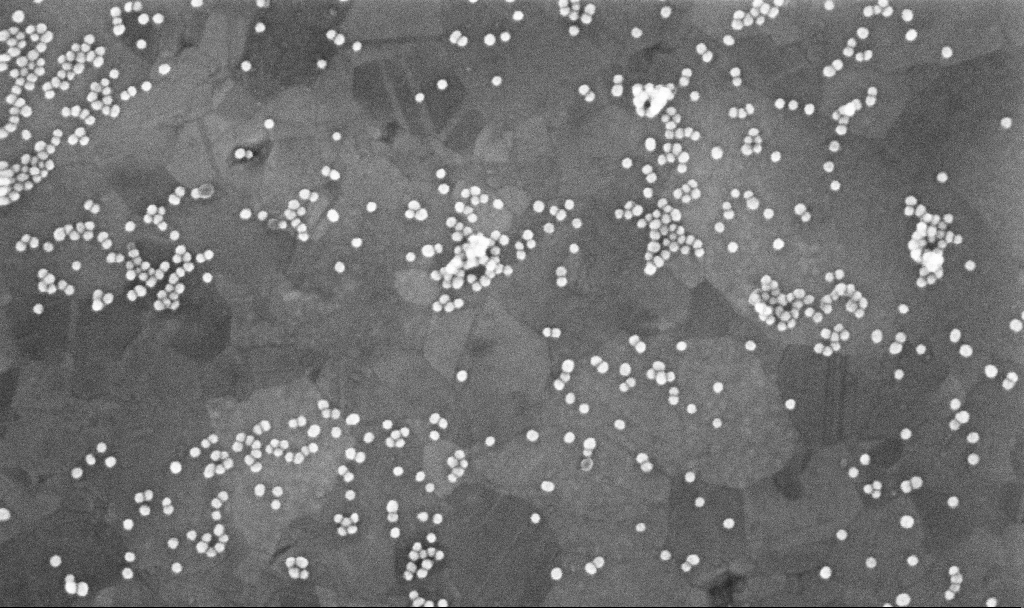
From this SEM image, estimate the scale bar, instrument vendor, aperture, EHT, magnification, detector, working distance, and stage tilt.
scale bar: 100 nm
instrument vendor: Zeiss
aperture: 30 µm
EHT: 10 kV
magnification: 200 K X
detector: InLens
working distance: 3.7 mm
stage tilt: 0°